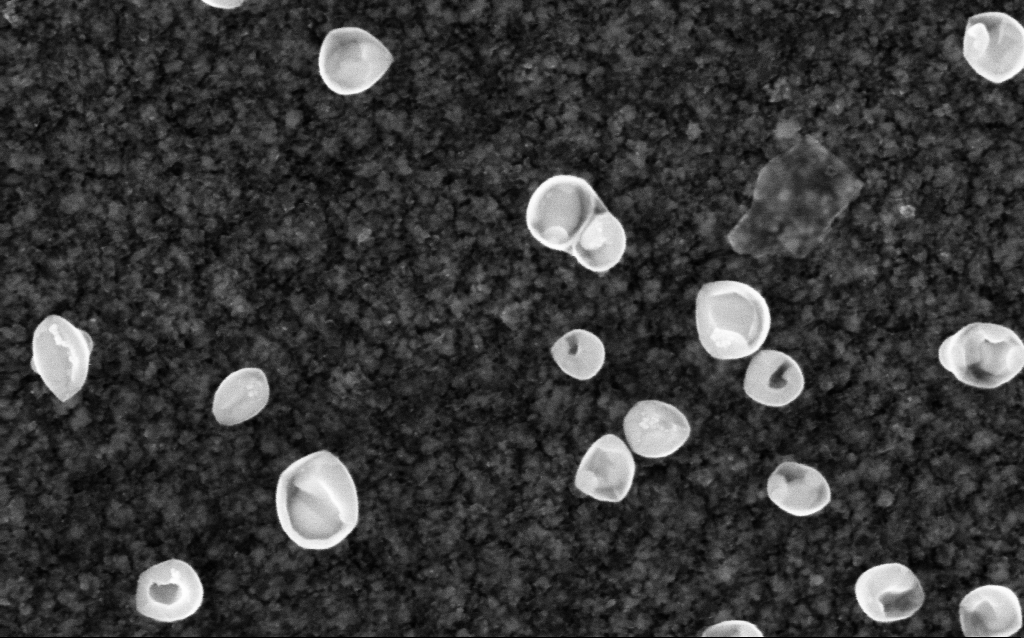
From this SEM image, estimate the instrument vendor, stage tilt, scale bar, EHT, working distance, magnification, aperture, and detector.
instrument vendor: Zeiss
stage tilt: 0°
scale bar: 100 nm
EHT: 5 kV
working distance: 2.8 mm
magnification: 200 K X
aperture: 30 µm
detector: InLens